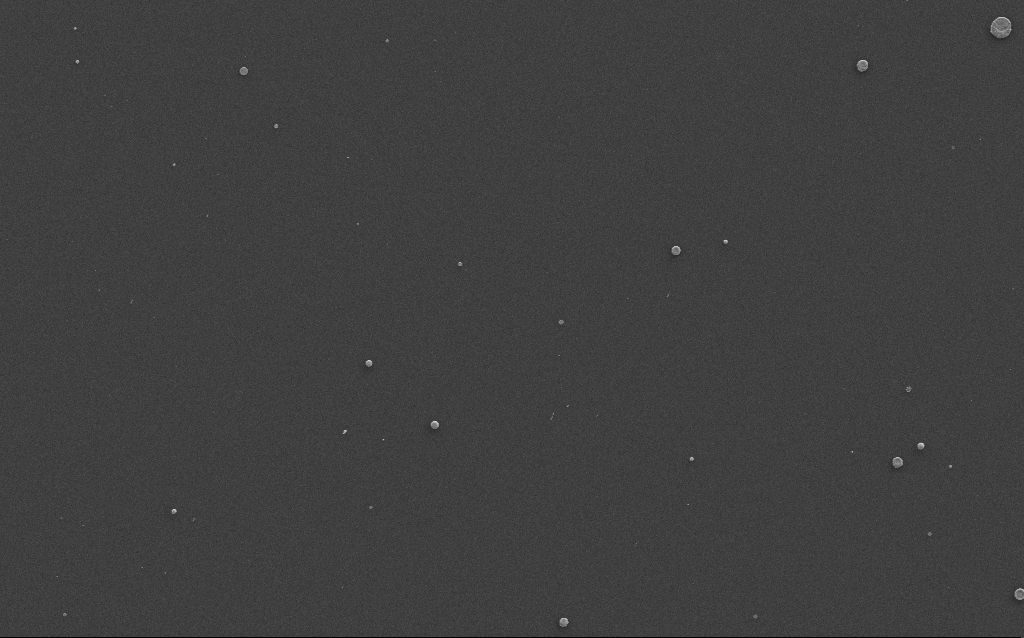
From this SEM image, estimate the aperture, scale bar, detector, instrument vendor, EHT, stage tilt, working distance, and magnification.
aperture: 30 µm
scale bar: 10000 nm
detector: SE2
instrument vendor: Zeiss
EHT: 10 kV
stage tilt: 0°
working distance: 3 mm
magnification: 3.51 K X